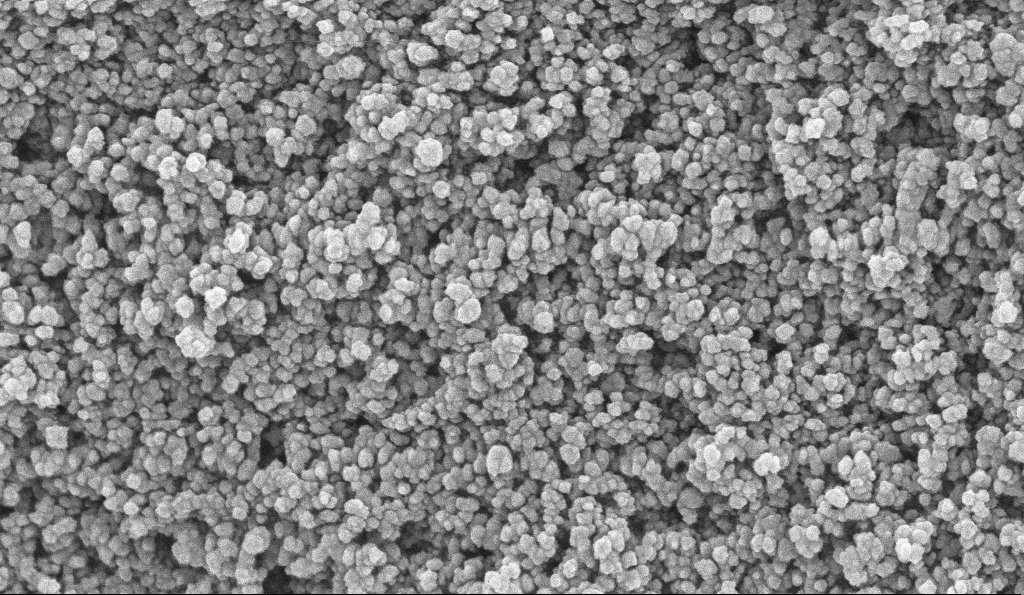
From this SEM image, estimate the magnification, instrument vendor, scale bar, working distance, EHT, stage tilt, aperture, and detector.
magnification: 135 K X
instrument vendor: Zeiss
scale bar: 100 nm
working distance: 5.1 mm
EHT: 10 kV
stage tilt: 0°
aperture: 30 µm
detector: InLens